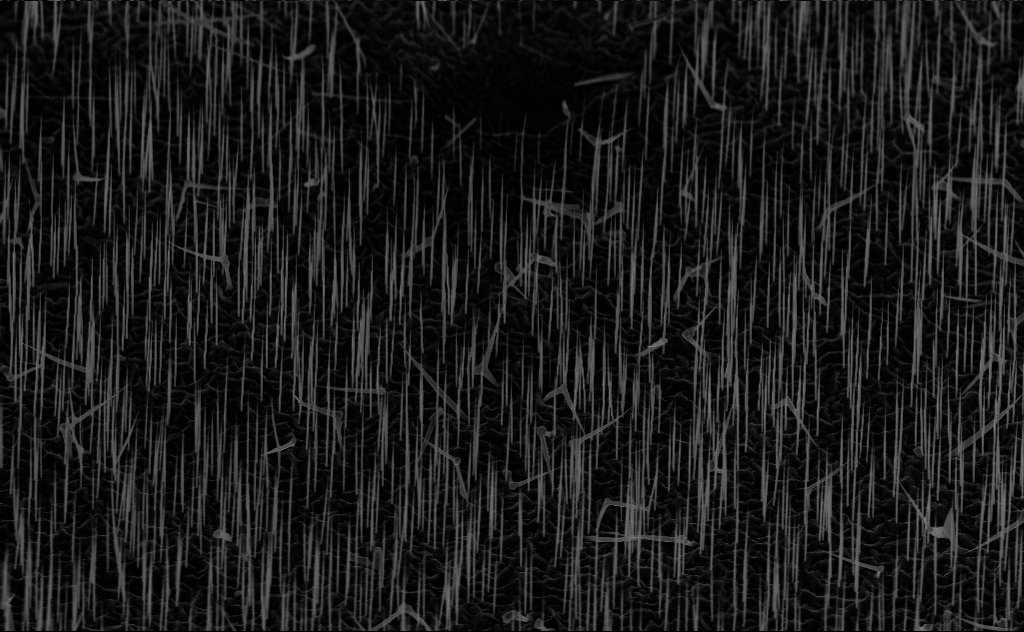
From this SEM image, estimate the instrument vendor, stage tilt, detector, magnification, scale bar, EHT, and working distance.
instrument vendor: Zeiss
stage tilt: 45°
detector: InLens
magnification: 5 K X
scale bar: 10000 nm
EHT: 10 kV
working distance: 7 mm